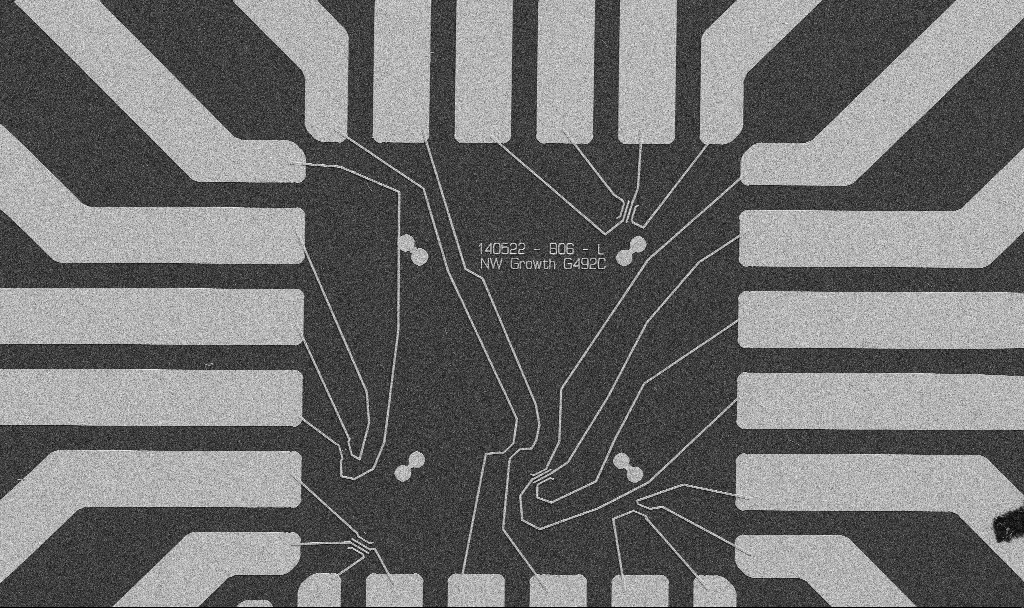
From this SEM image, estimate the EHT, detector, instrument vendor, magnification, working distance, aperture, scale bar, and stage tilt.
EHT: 5 kV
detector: SE2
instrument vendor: Zeiss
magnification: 1 K X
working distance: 10.7 mm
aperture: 30 µm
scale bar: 20000 nm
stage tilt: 0°